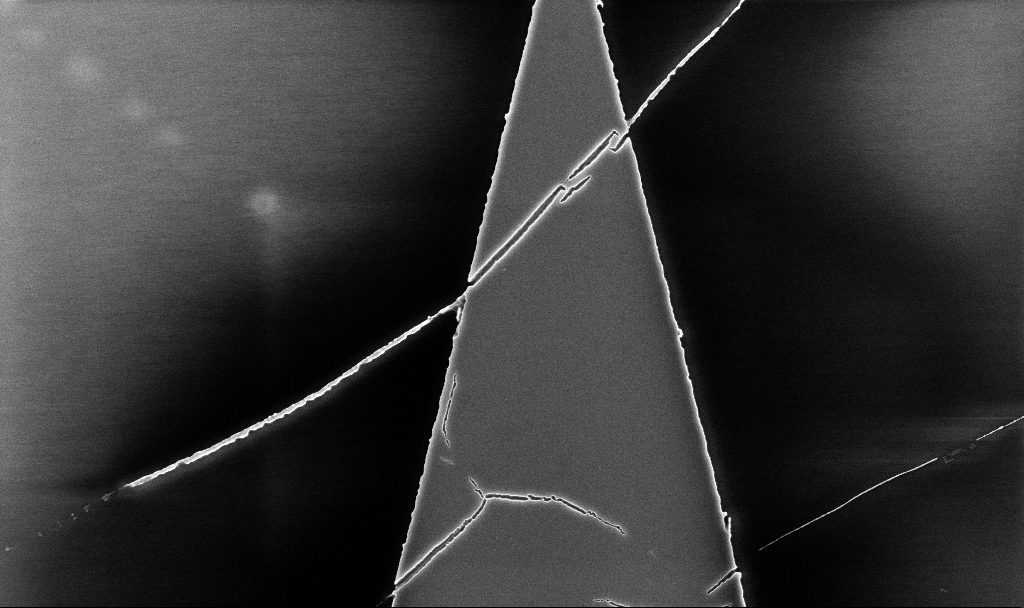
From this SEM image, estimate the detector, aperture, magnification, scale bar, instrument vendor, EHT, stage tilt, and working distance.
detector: InLens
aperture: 30 µm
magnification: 12.37 K X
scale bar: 2000 nm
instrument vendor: Zeiss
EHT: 5 kV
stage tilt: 0°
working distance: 5.2 mm